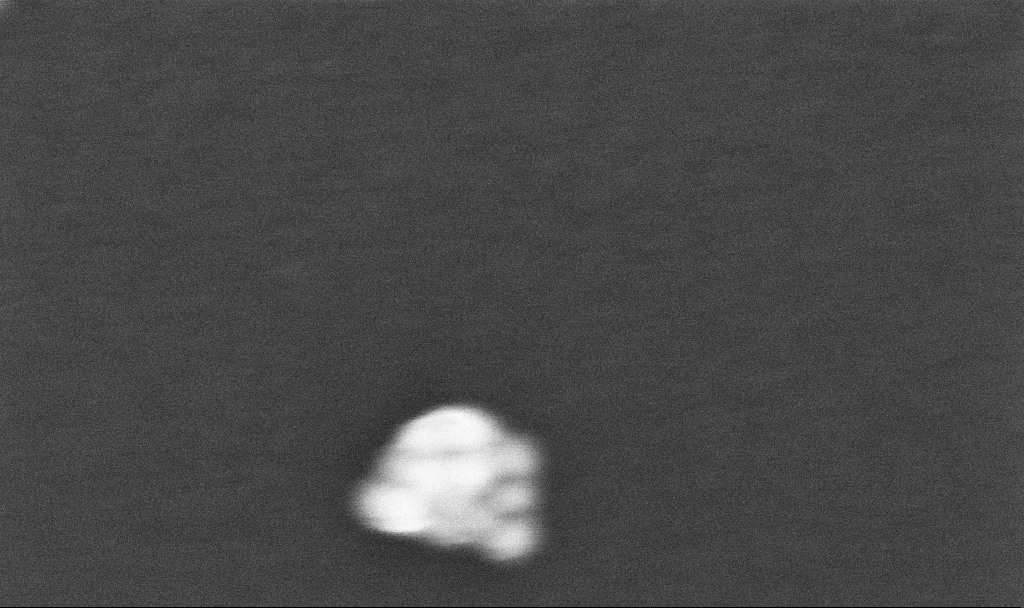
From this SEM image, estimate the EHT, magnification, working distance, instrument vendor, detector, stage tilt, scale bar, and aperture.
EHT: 10 kV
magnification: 600 K X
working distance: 3.1 mm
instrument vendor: Zeiss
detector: InLens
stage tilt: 0°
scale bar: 100 nm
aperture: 30 µm